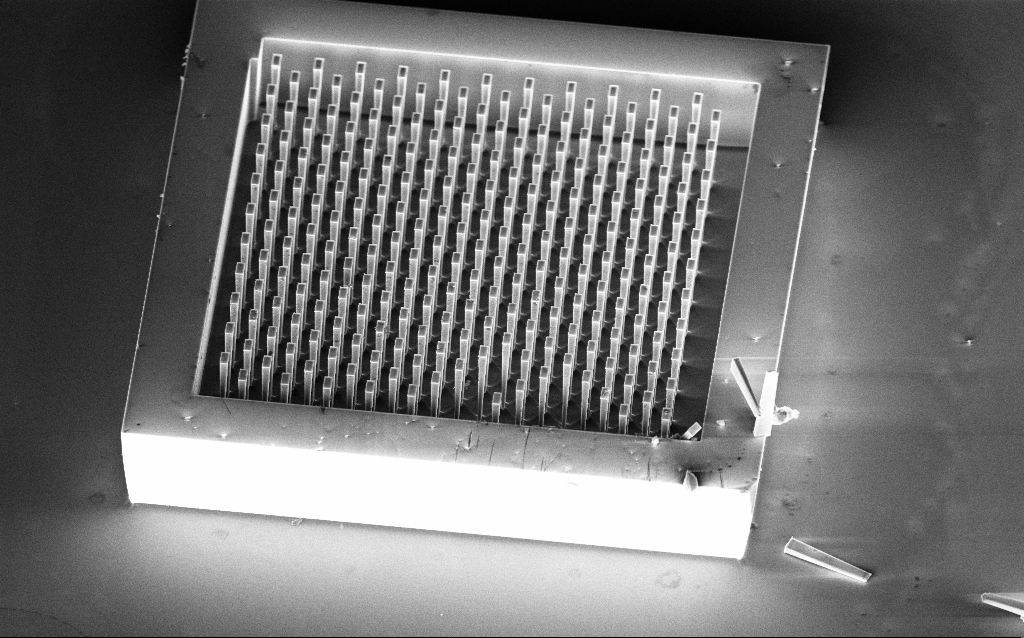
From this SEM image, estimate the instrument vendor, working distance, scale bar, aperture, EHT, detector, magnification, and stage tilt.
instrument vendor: Zeiss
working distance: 8 mm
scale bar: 20000 nm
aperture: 30 µm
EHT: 10 kV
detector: InLens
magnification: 0.779 K X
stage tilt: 45°